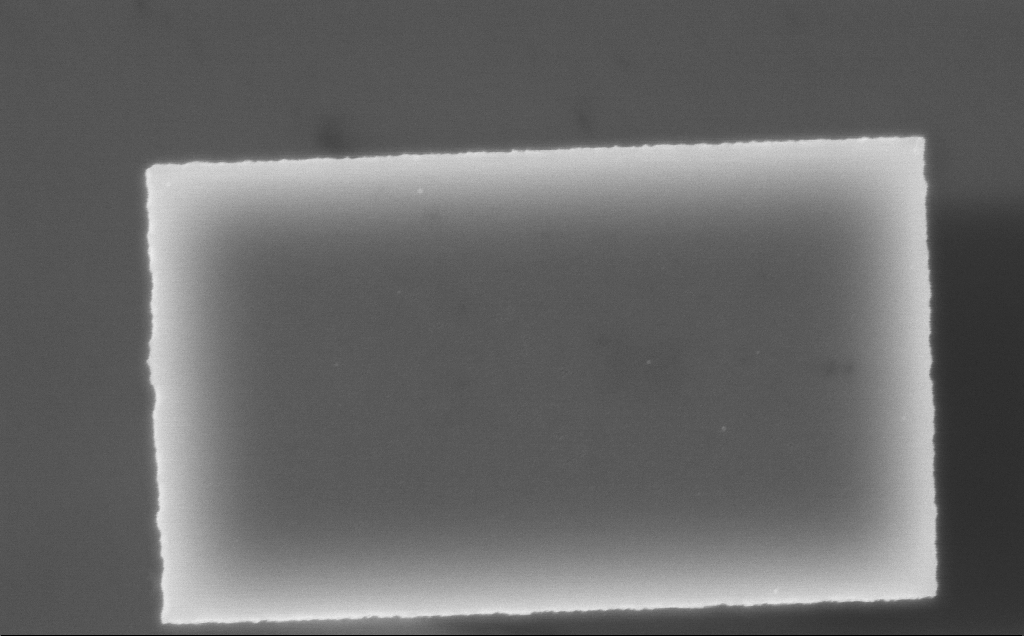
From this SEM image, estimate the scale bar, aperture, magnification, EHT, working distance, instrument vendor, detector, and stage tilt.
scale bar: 1000 nm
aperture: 30 µm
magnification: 59.45 K X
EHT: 7.5 kV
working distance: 4 mm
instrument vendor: Zeiss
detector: InLens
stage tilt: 0°